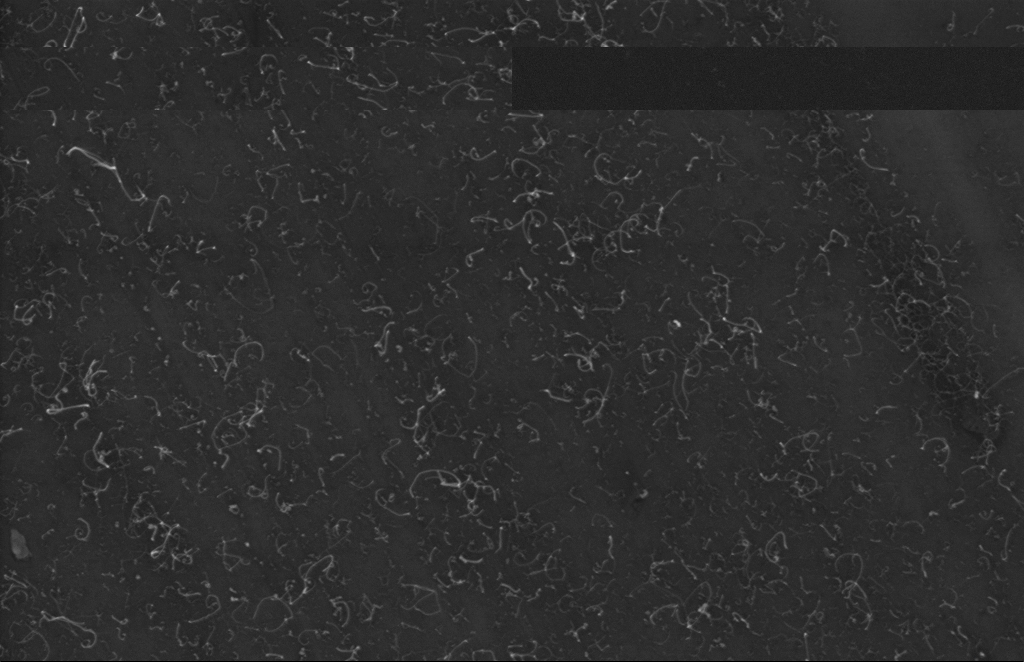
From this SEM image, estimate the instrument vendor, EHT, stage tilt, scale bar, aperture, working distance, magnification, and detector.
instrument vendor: Zeiss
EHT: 5 kV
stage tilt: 0°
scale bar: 200 nm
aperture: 20 µm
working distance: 5 mm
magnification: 47.74 K X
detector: InLens